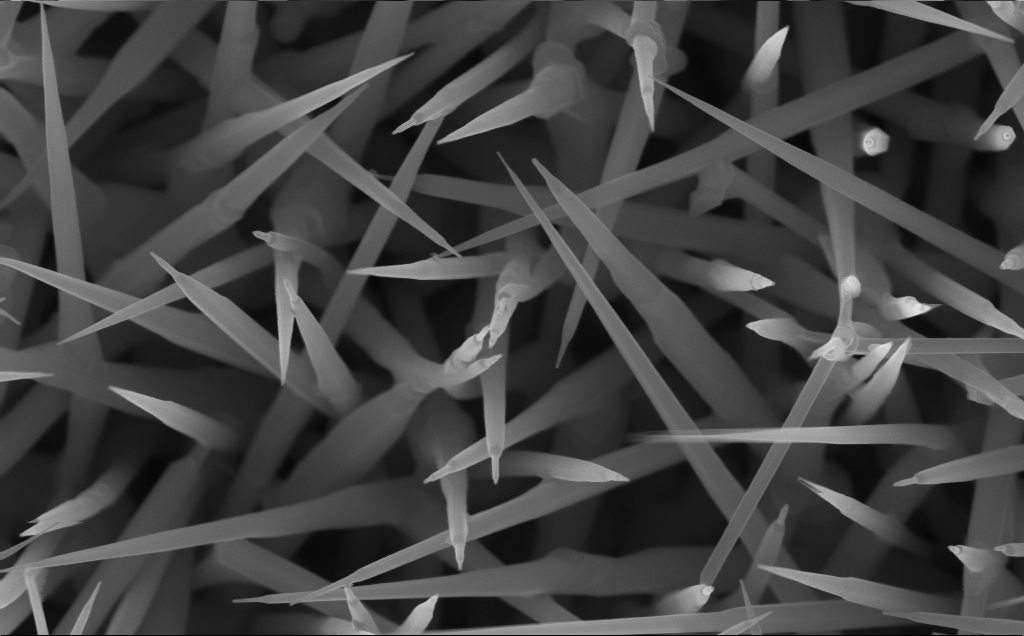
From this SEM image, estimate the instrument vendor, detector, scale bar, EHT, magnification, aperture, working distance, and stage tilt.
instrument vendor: Zeiss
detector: InLens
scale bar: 200 nm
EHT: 10 kV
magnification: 80 K X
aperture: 30 µm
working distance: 5 mm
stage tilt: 0°